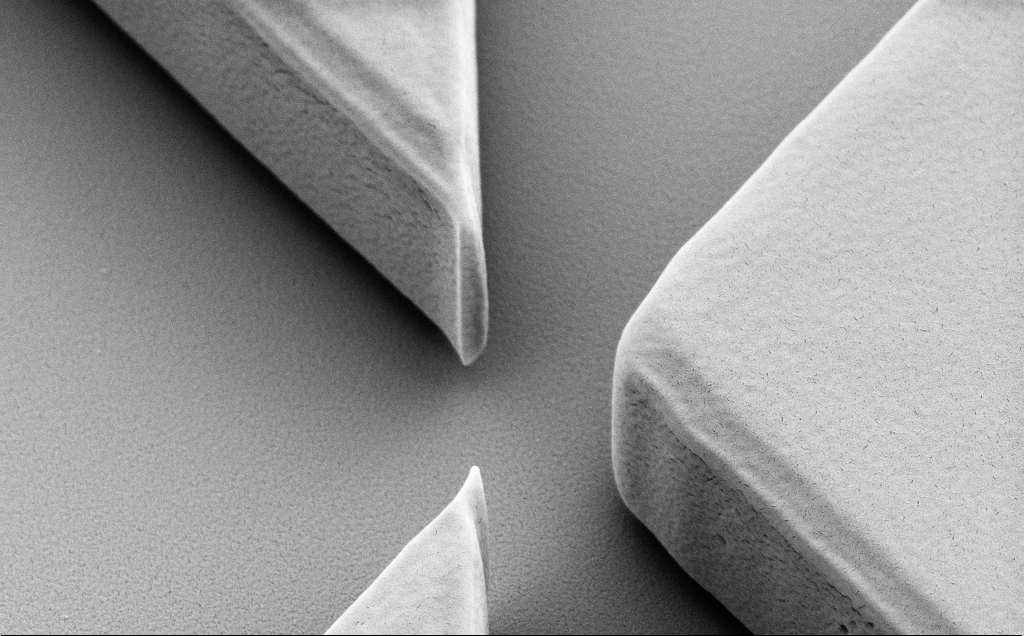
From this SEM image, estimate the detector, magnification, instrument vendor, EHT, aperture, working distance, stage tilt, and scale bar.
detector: SE2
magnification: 17.41 K X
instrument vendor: Zeiss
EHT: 5 kV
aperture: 30 µm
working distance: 9 mm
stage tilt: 40°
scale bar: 2000 nm